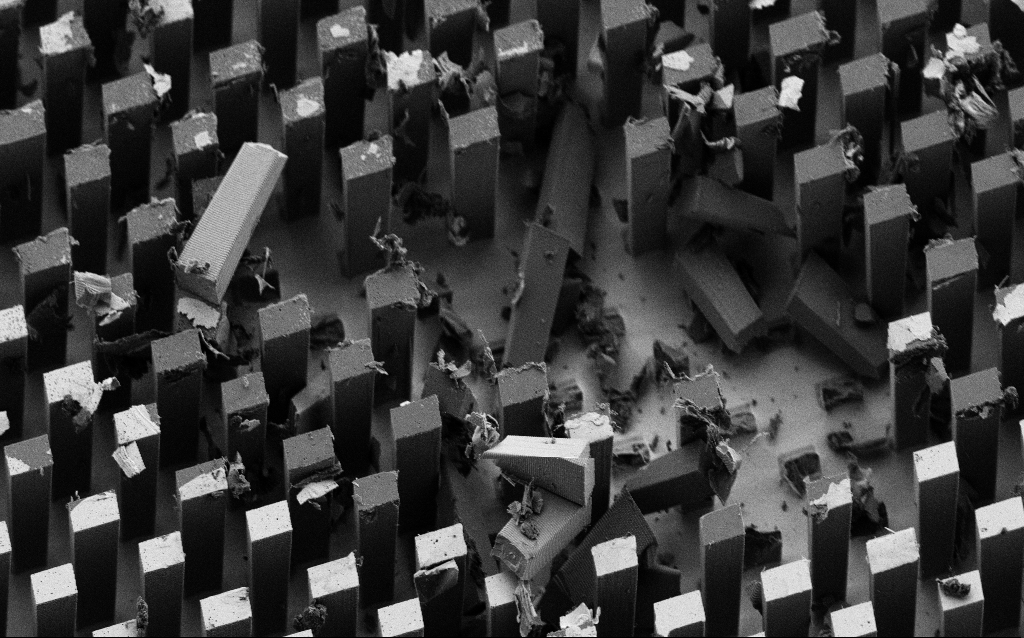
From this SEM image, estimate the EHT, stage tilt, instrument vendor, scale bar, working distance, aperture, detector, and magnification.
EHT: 2.5 kV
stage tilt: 30.2°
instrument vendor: Zeiss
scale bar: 10000 nm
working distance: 8 mm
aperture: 30 µm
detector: SE2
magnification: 2.01 K X